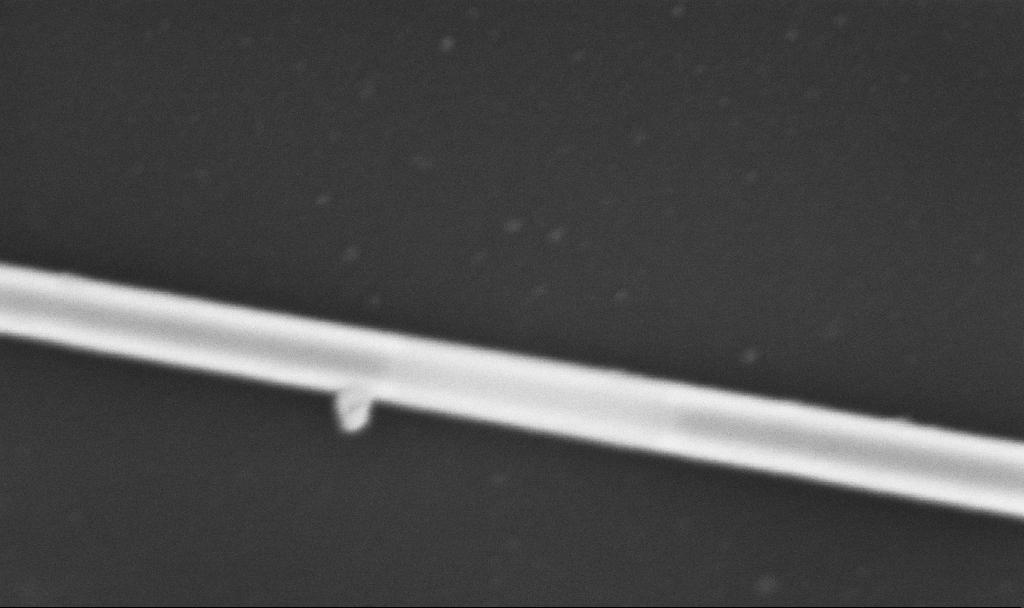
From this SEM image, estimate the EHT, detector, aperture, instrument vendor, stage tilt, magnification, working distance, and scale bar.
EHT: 5 kV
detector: InLens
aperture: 30 µm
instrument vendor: Zeiss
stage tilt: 0°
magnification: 300 K X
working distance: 6.7 mm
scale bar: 200 nm